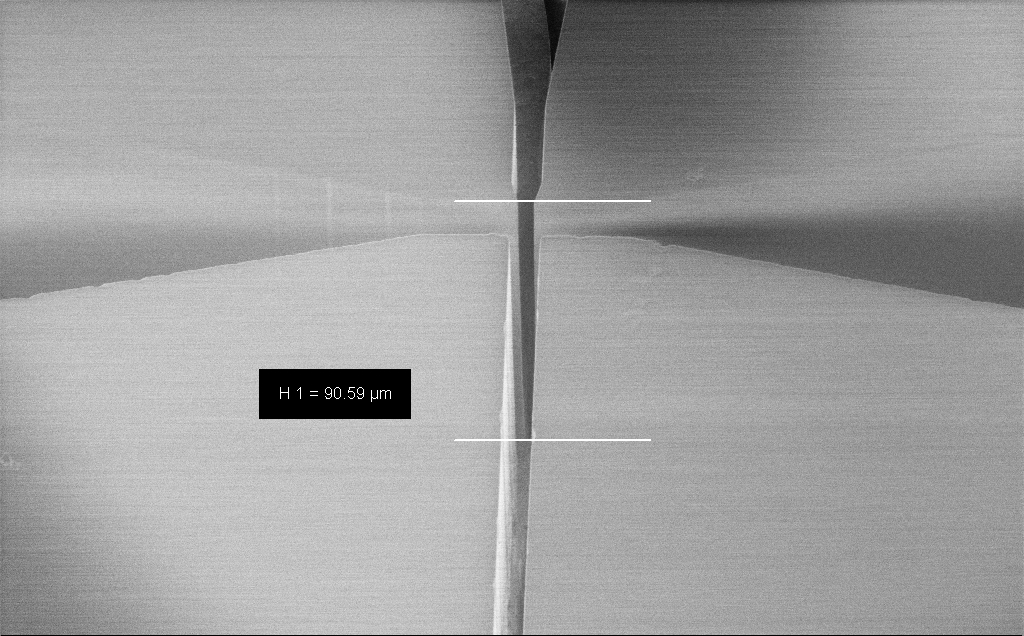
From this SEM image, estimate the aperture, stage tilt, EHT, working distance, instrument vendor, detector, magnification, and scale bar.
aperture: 30 µm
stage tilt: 45°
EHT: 1.2 kV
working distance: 6 mm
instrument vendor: Zeiss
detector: InLens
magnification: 0.969 K X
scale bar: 20000 nm